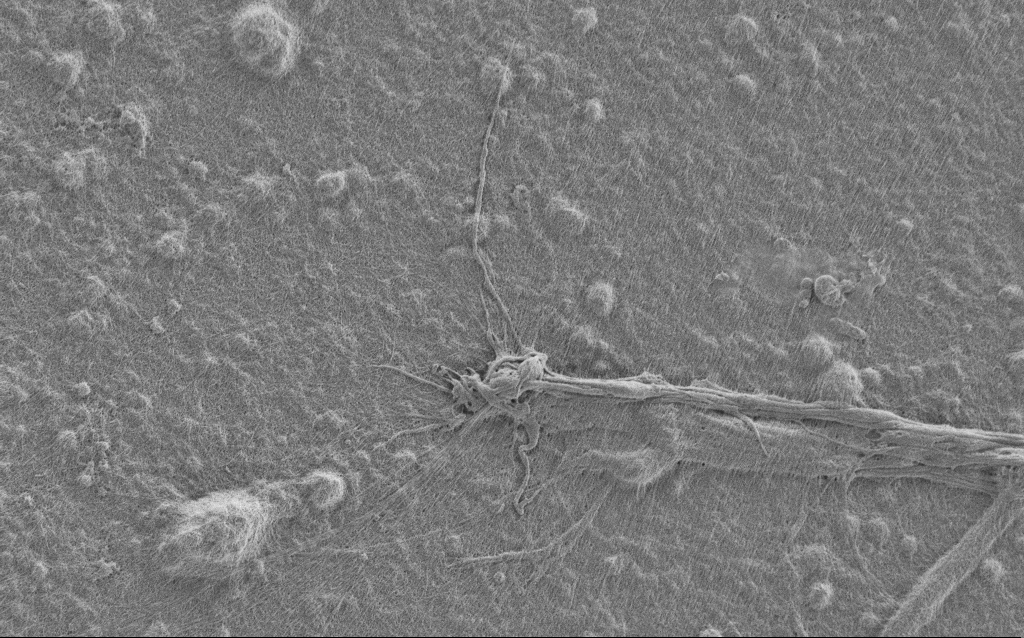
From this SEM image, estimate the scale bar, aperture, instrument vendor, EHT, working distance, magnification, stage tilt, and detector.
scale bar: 2000 nm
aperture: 30 µm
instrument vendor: Zeiss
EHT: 1 kV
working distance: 6 mm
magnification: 7.5 K X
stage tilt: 0°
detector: SE2